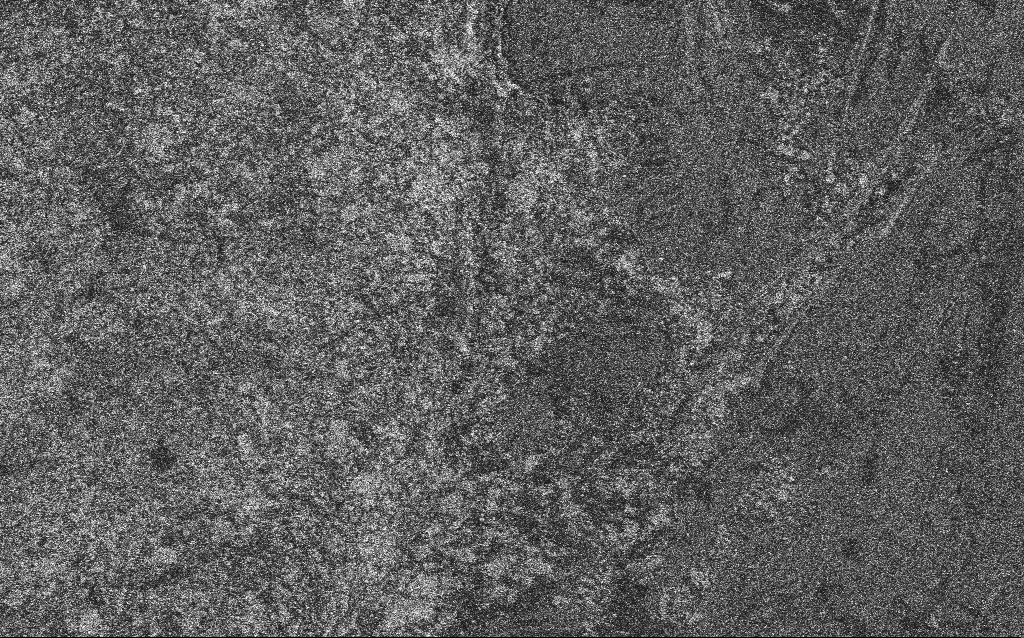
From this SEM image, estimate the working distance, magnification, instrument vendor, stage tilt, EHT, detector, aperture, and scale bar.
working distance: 6 mm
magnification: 0.5 K X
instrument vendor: Zeiss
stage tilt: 0°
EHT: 3 kV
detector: SE2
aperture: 30 µm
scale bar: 100000 nm